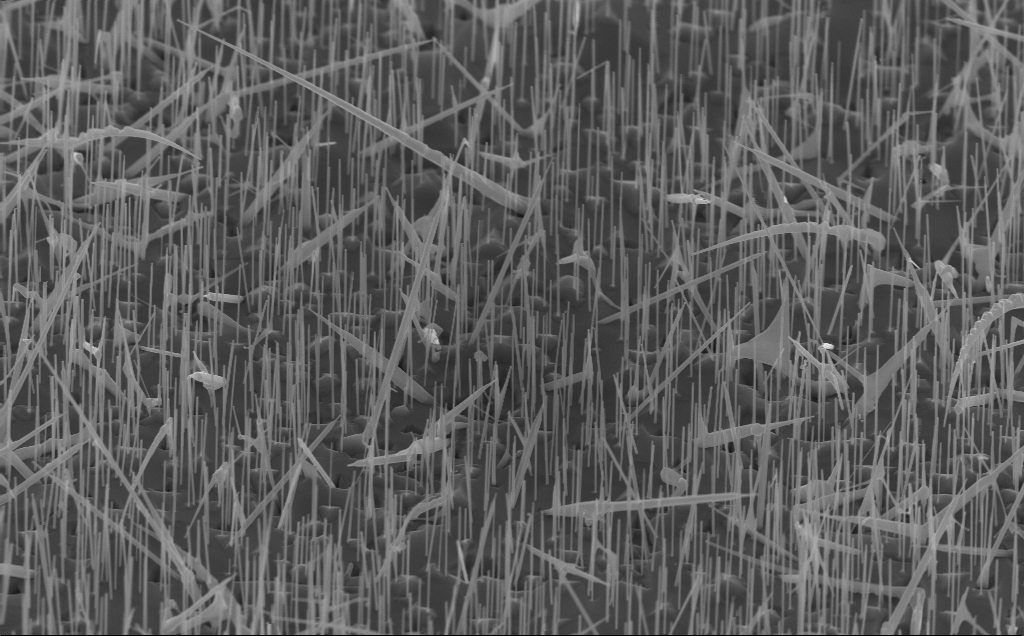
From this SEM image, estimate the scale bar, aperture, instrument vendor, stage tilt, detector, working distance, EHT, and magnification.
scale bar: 1000 nm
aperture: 30 µm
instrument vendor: Zeiss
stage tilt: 45°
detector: InLens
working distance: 5 mm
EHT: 10 kV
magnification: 20 K X